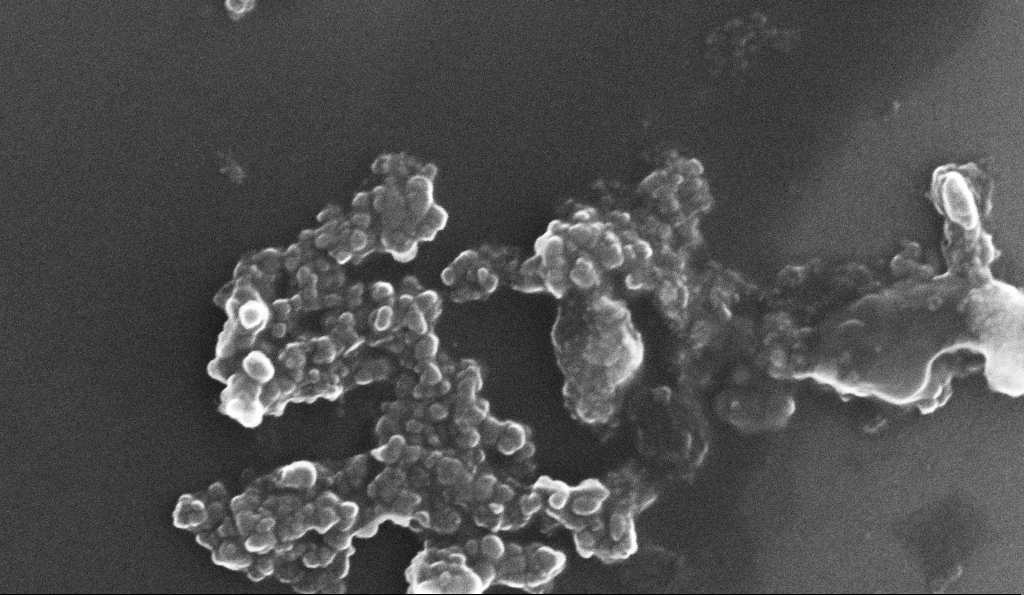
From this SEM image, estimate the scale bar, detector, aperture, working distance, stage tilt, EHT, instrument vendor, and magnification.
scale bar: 200 nm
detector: InLens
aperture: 30 µm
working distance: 5.3 mm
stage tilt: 0°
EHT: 10 kV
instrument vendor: Zeiss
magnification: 172.24 K X